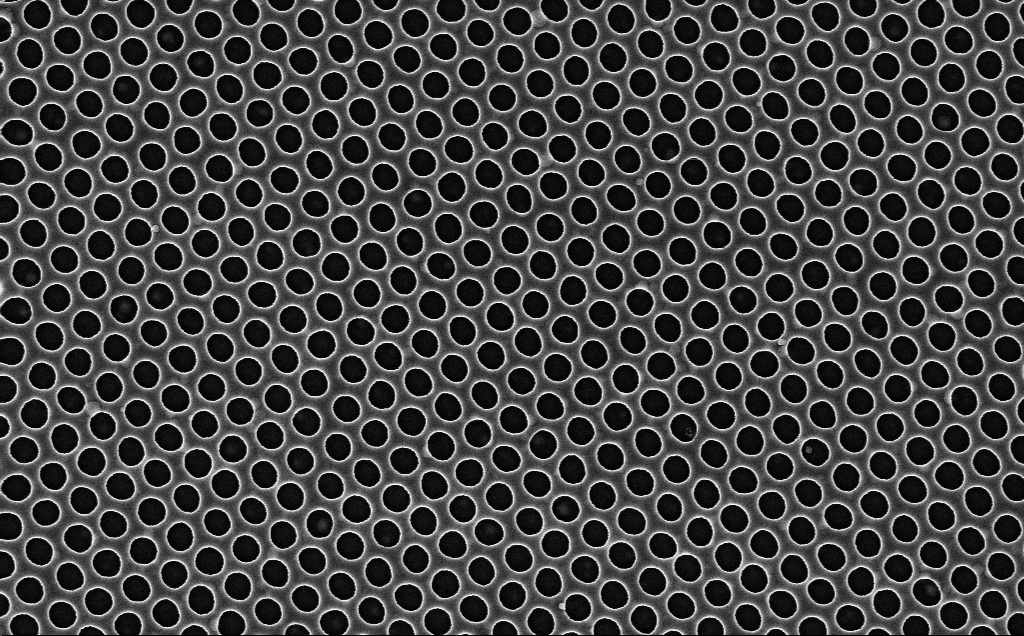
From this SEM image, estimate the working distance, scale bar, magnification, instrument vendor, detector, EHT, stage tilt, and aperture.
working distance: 2.4 mm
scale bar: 1000 nm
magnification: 40 K X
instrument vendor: Zeiss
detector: InLens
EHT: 3 kV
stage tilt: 0°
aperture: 30 µm